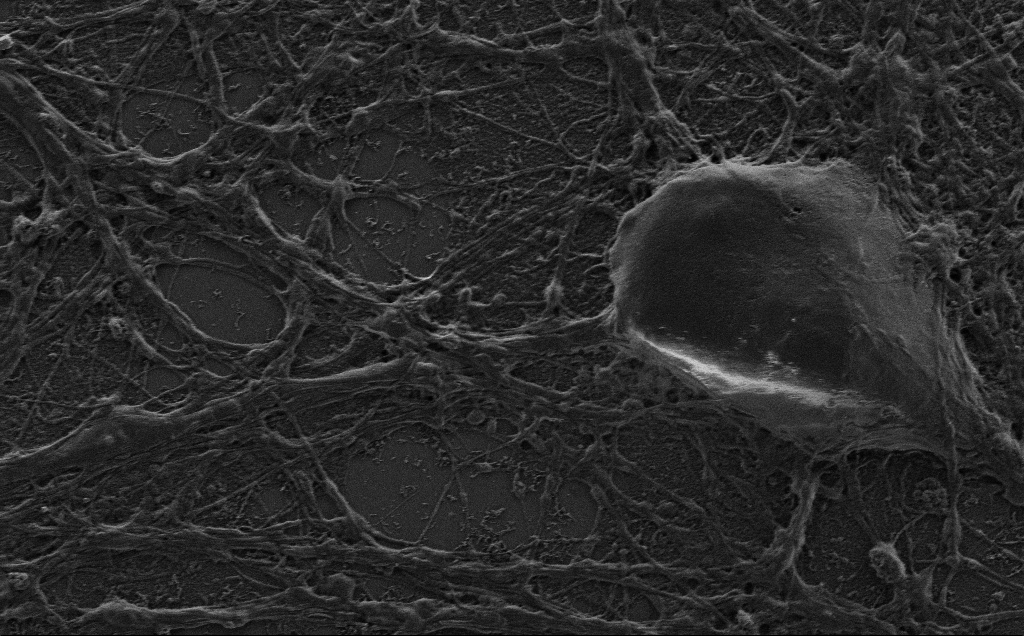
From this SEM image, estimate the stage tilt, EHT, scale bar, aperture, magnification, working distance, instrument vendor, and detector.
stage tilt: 0°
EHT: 1.5 kV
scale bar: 2000 nm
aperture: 30 µm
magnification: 7.57 K X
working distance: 4 mm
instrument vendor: Zeiss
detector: SE2